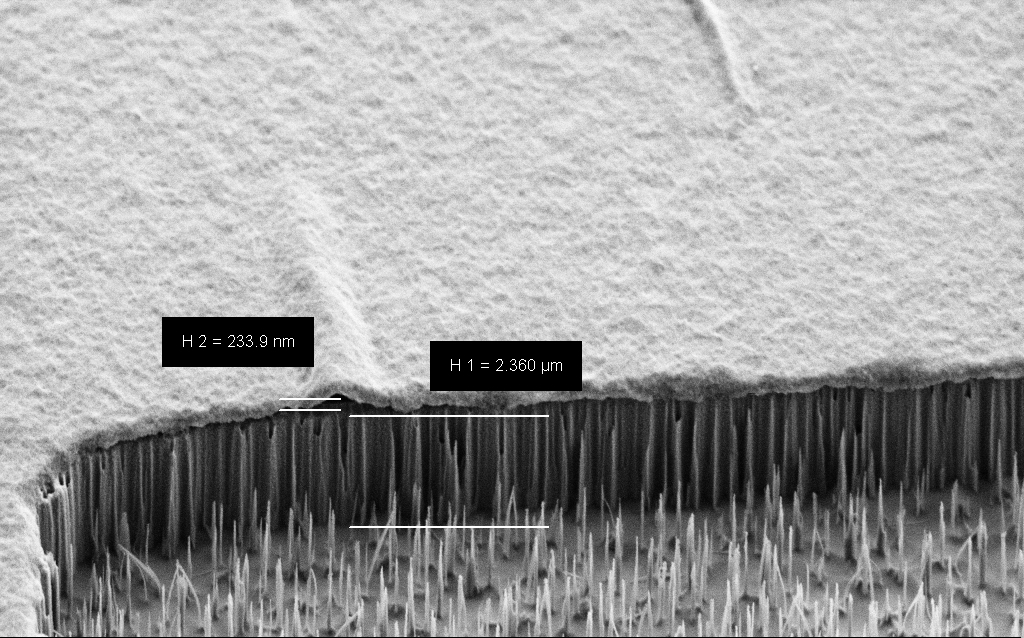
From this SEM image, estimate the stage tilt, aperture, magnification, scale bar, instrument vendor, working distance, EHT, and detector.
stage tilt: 45°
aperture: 30 µm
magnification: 17.27 K X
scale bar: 1000 nm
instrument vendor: Zeiss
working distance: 7 mm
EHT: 2 kV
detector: SE2